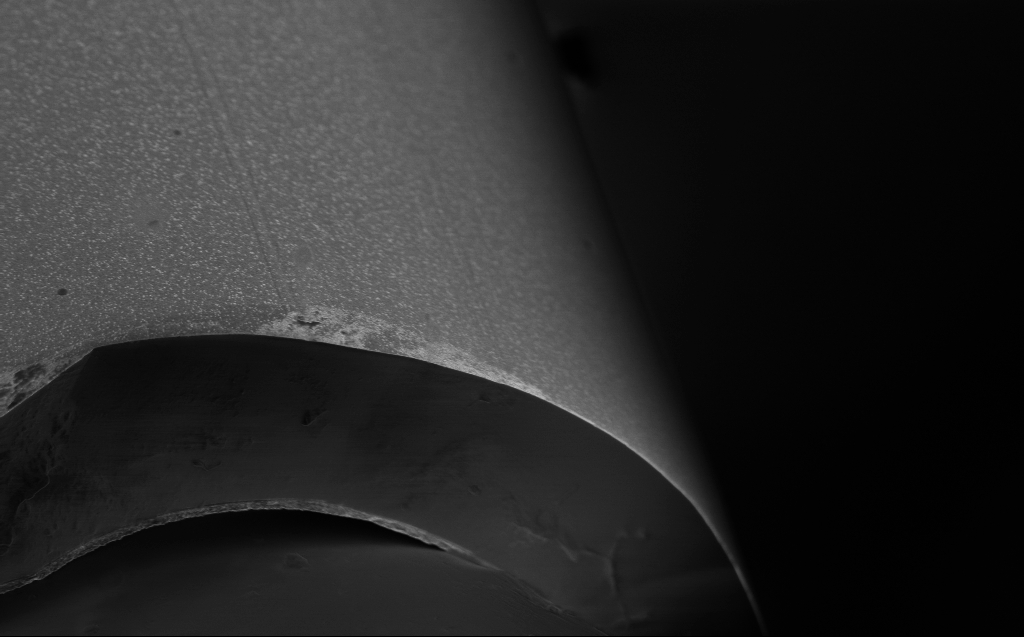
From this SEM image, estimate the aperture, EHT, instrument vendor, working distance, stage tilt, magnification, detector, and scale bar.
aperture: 30 µm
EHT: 1 kV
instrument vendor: Zeiss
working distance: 3 mm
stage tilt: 45°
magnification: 8.2 K X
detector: InLens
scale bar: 2000 nm